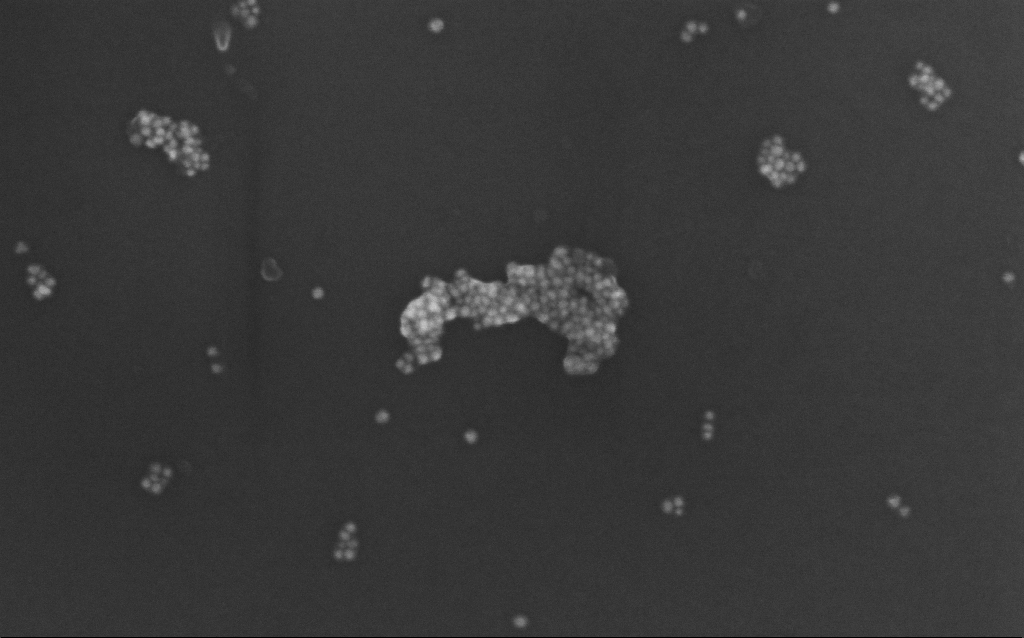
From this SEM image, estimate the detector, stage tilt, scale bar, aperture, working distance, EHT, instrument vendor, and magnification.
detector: InLens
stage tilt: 0°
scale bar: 200 nm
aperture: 30 µm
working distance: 7 mm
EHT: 10 kV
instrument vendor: Zeiss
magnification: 216.81 K X